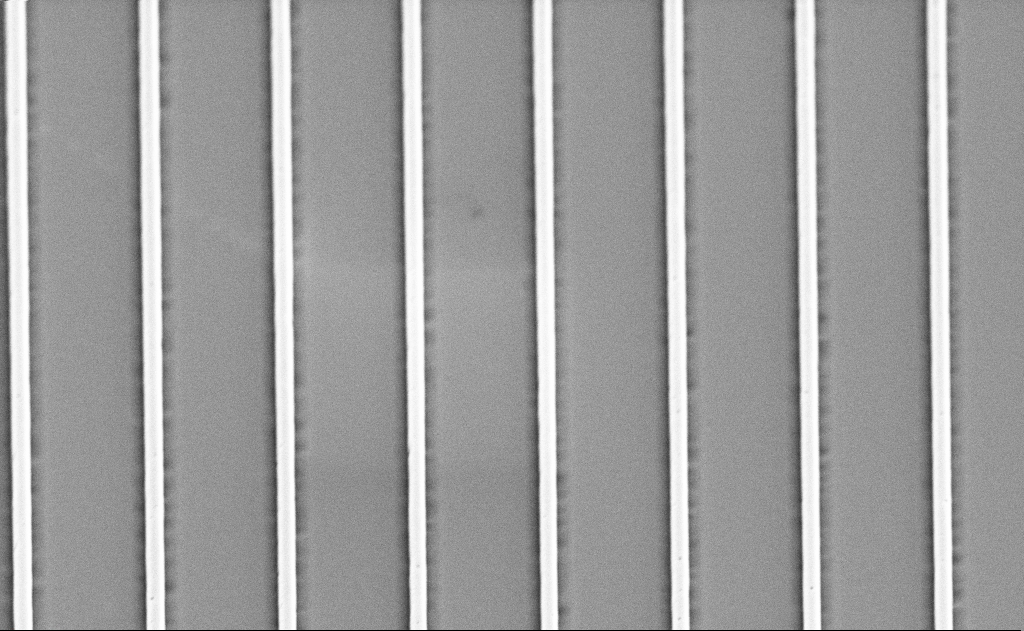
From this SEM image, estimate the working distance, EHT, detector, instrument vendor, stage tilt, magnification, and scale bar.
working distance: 11 mm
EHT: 5 kV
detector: SE2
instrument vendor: Zeiss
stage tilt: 45°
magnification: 12.04 K X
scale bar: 2000 nm